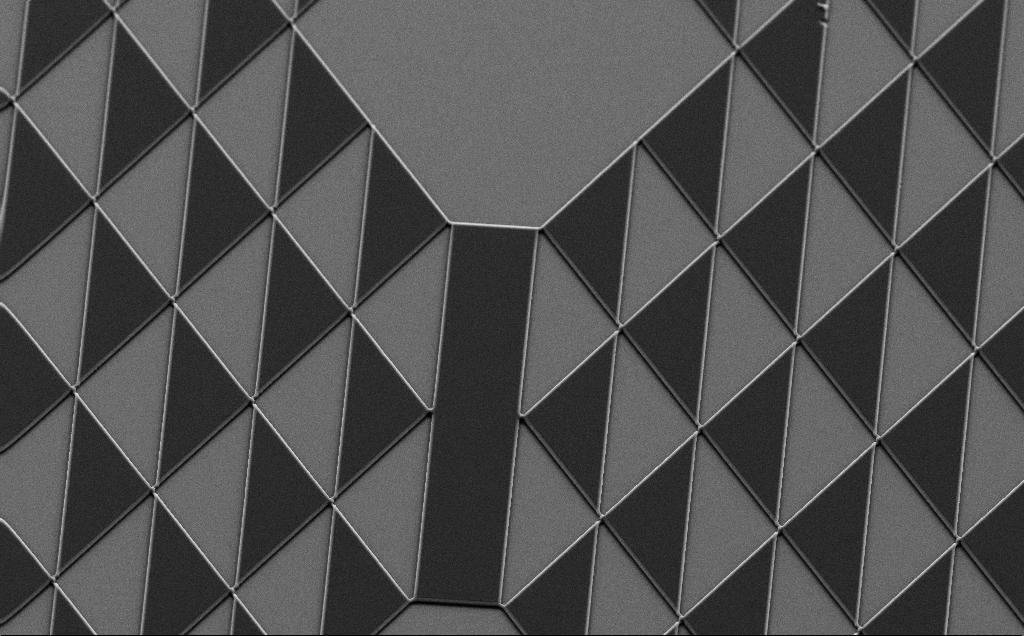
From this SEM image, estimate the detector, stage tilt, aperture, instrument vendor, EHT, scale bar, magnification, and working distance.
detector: SE2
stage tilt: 35°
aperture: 30 µm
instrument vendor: Zeiss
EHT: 10 kV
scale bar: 20000 nm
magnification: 0.933 K X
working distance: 8 mm